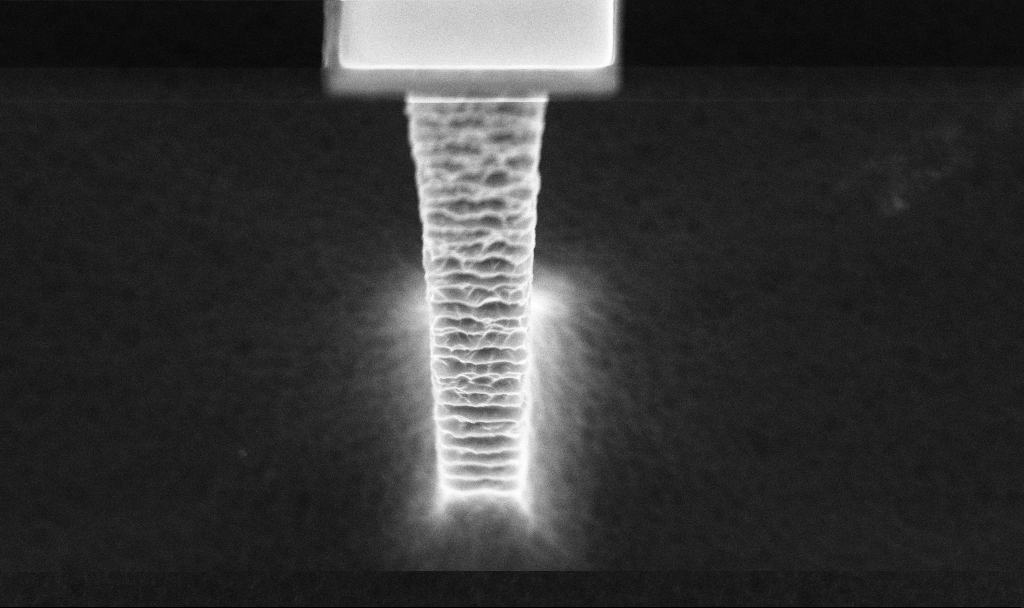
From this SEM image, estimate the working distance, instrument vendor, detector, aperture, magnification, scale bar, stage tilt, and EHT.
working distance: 4.2 mm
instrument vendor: Zeiss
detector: InLens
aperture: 30 µm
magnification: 36.2 K X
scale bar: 2000 nm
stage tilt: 20°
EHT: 5 kV